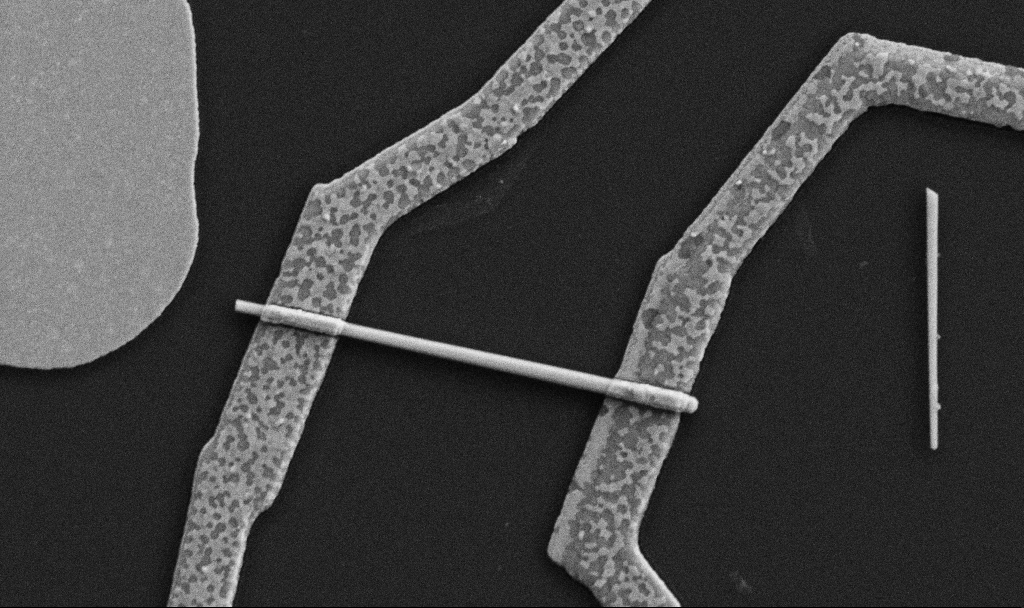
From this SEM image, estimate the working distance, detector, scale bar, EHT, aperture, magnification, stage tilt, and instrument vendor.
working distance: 8.7 mm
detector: SE2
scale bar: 1000 nm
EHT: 5 kV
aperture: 30 µm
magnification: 30 K X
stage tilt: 0°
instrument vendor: Zeiss